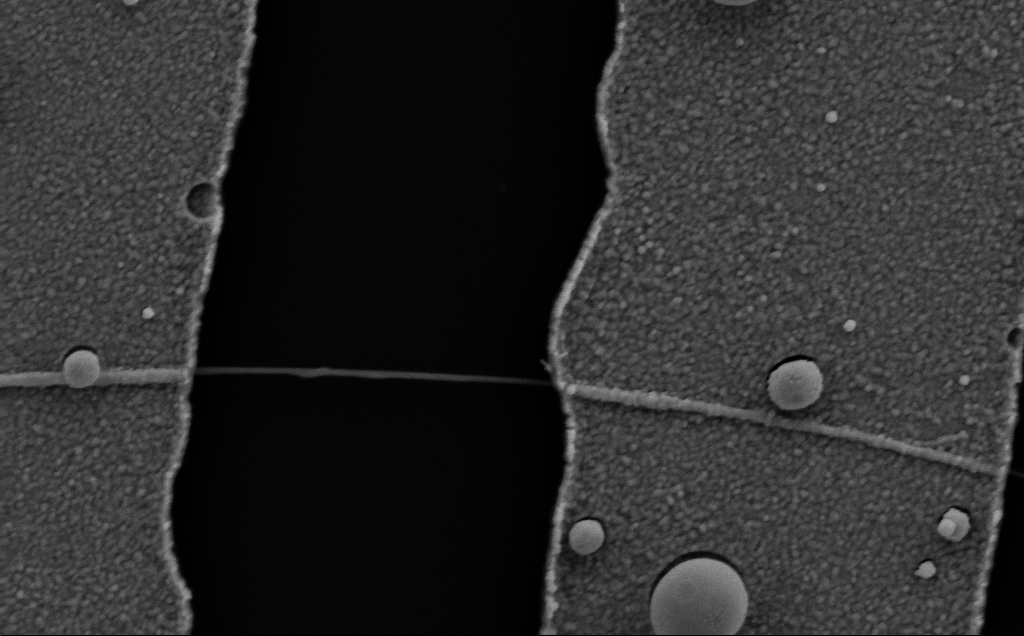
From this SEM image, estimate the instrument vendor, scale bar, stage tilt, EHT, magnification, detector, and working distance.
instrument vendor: Zeiss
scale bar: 200 nm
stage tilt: -0.7°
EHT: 5 kV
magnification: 75.53 K X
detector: SE2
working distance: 8 mm